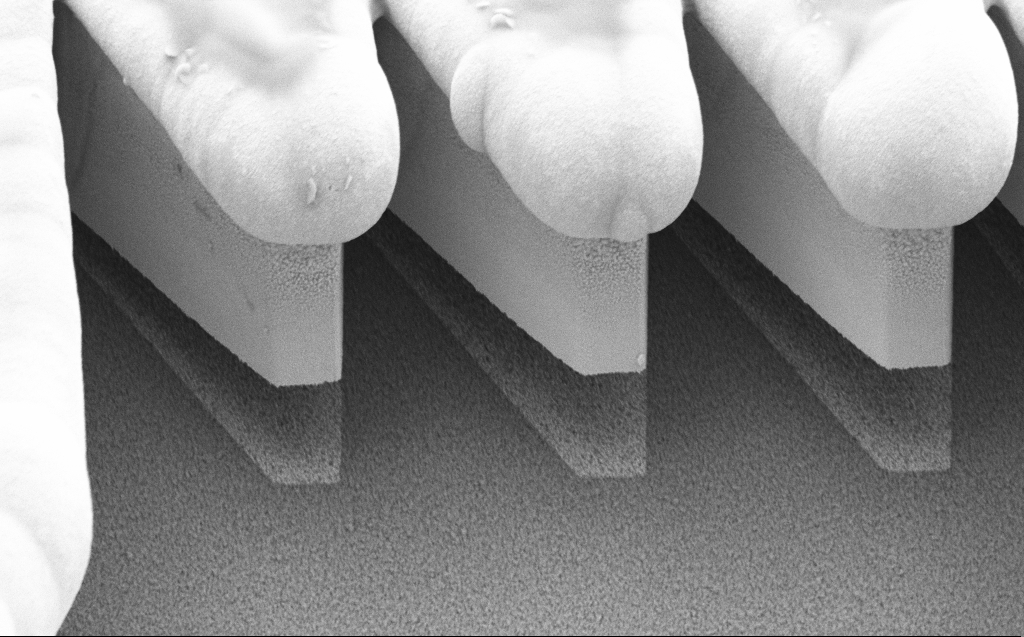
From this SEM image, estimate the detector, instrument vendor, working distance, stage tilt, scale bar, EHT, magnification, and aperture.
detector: SE2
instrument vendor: Zeiss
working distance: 9 mm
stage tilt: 45°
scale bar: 2000 nm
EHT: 5 kV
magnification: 9.81 K X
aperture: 30 µm